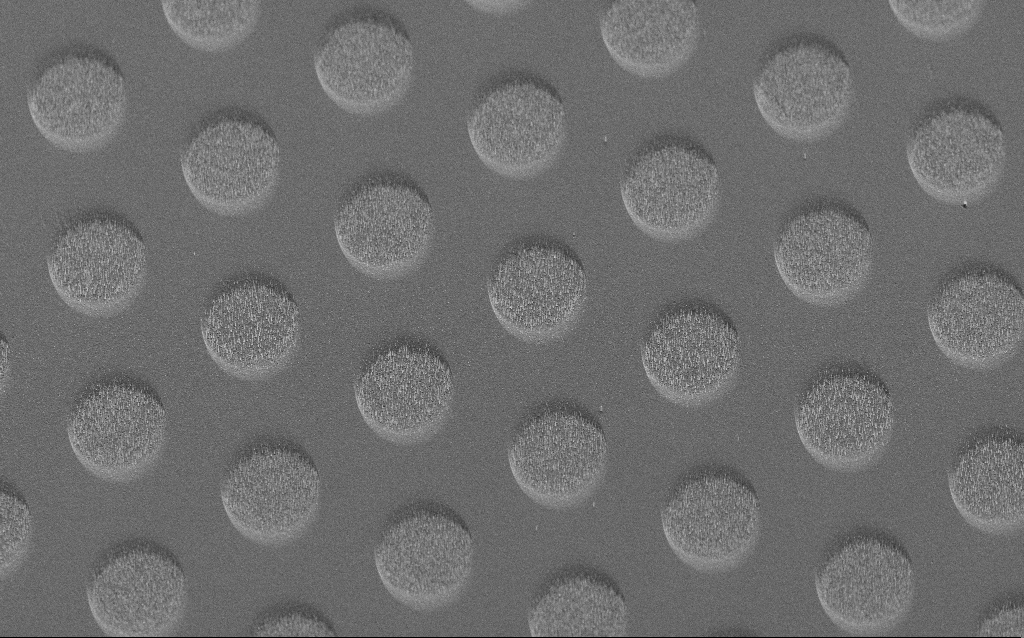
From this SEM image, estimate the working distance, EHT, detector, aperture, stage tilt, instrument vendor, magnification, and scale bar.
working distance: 8 mm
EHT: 5 kV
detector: SE2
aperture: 30 µm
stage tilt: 45°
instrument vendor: Zeiss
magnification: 0.882 K X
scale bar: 20000 nm